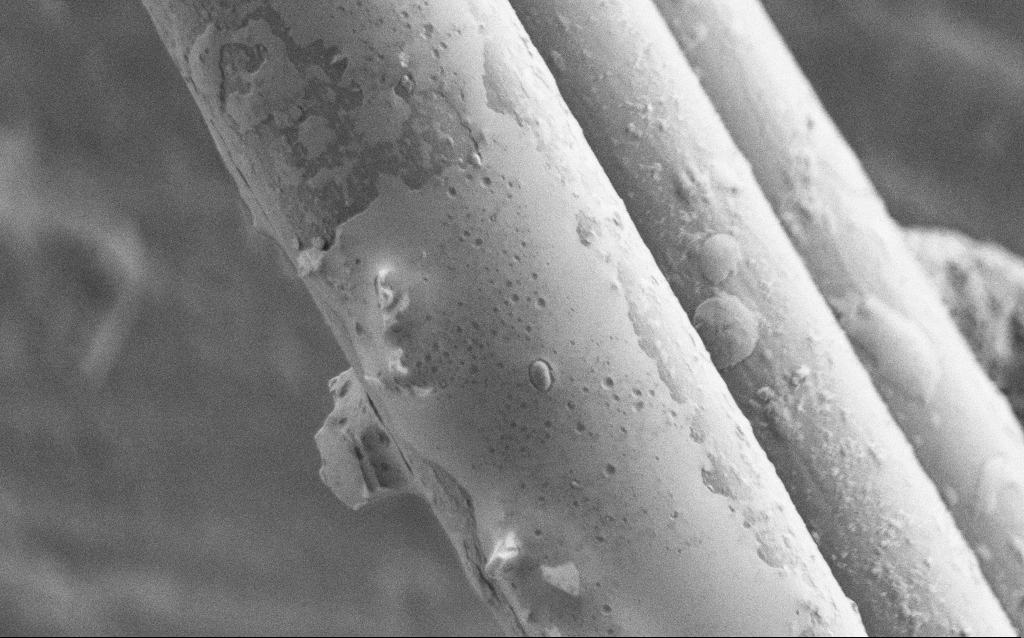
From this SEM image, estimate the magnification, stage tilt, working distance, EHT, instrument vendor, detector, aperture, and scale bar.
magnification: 5.19 K X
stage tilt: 0°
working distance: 5 mm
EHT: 1 kV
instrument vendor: Zeiss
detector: SE2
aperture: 30 µm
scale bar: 10000 nm